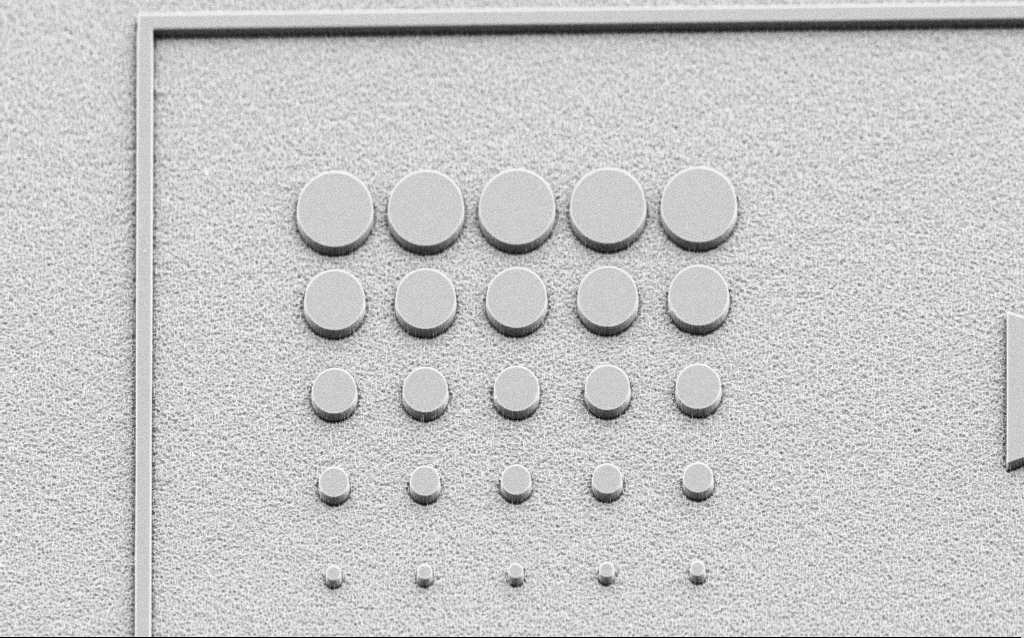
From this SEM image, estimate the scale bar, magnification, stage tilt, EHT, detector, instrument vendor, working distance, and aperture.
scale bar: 10000 nm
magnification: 5.56 K X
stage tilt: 45°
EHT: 3 kV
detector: SE2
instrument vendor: Zeiss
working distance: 8 mm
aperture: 30 µm